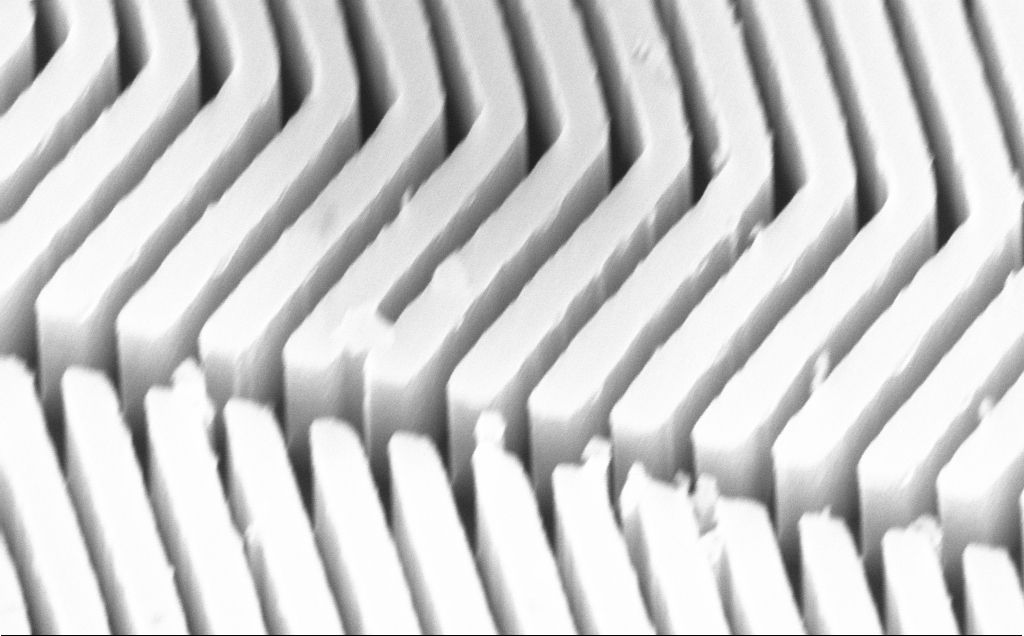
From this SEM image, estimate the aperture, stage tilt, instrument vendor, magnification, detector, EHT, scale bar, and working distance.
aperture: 30 µm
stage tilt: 45°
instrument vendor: Zeiss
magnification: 102.82 K X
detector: InLens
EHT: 10 kV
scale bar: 200 nm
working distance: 6 mm